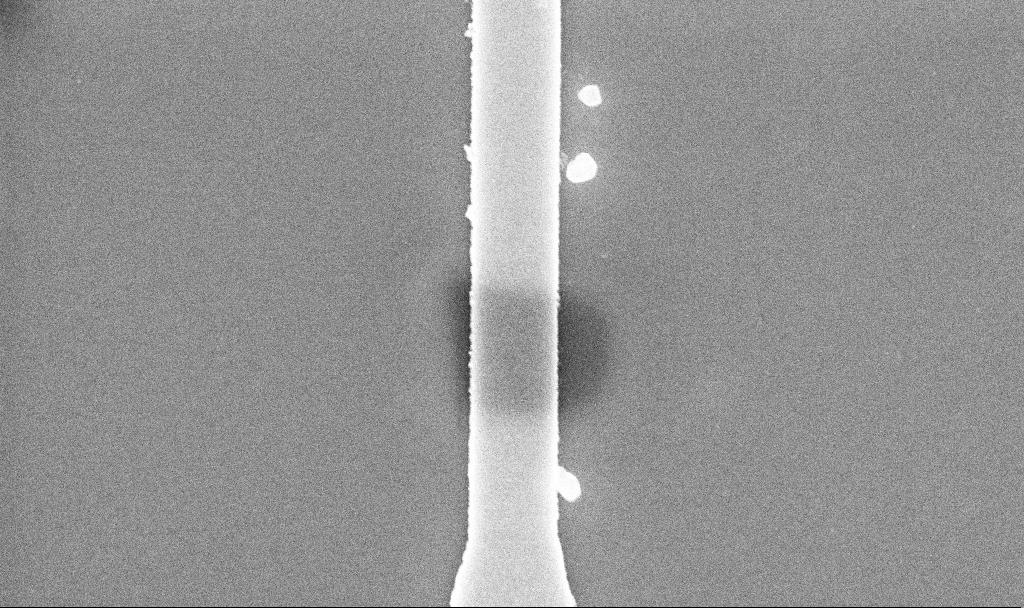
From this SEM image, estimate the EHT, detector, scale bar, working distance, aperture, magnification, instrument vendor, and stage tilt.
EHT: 5 kV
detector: InLens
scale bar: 1000 nm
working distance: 10.3 mm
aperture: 30 µm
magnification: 61.1 K X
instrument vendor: Zeiss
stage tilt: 0°